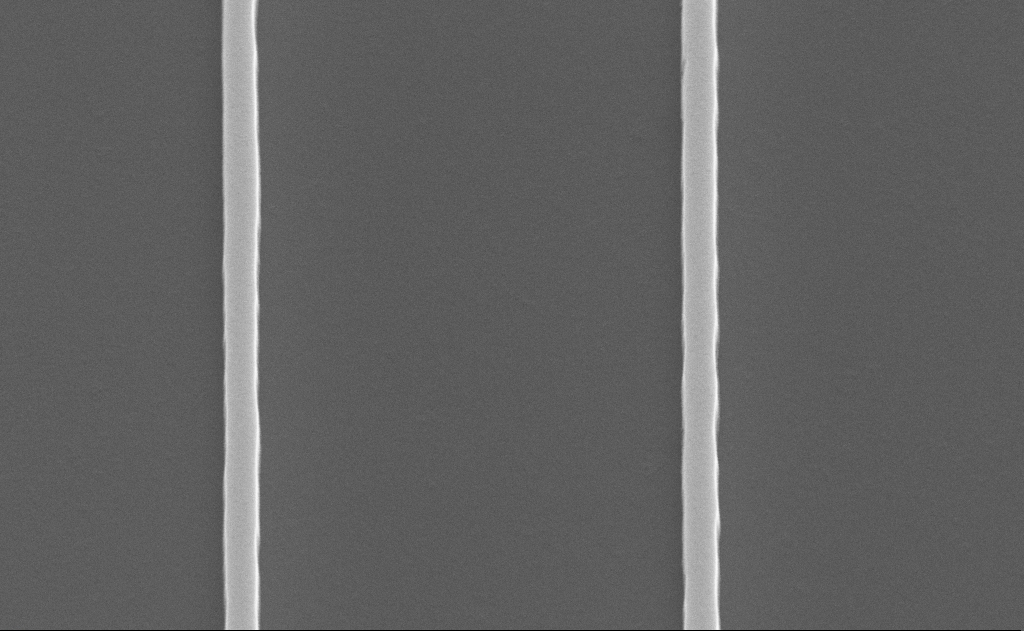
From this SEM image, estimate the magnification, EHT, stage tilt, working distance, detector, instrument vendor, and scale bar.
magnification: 41.94 K X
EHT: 10 kV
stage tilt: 50°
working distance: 12 mm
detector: SE2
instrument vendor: Zeiss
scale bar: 1000 nm